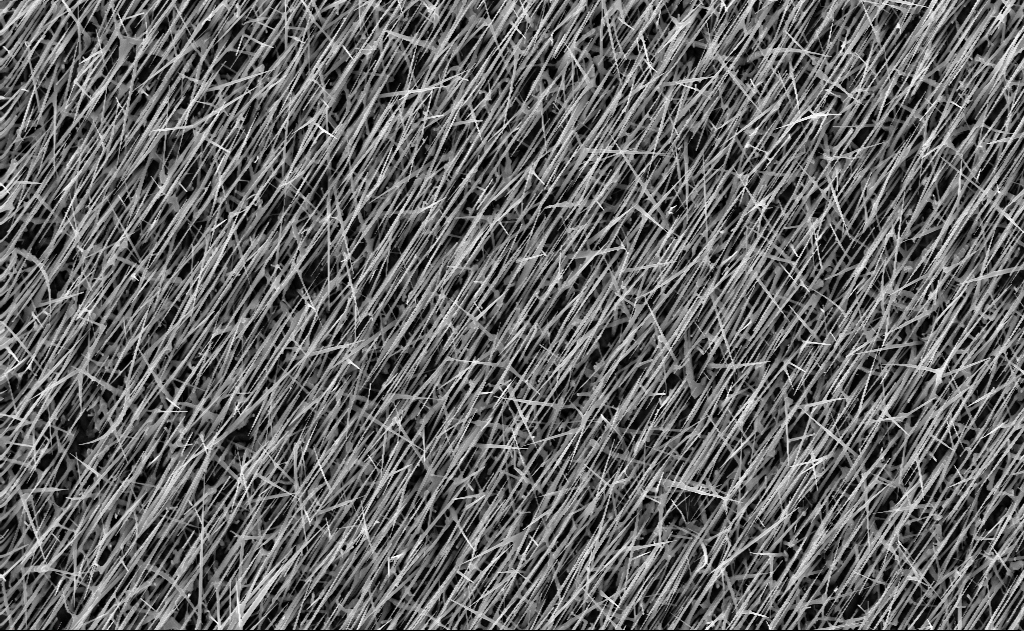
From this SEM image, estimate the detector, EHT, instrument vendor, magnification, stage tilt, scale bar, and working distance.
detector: InLens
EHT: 10 kV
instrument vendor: Zeiss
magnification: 10 K X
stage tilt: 0°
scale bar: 2000 nm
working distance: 7 mm